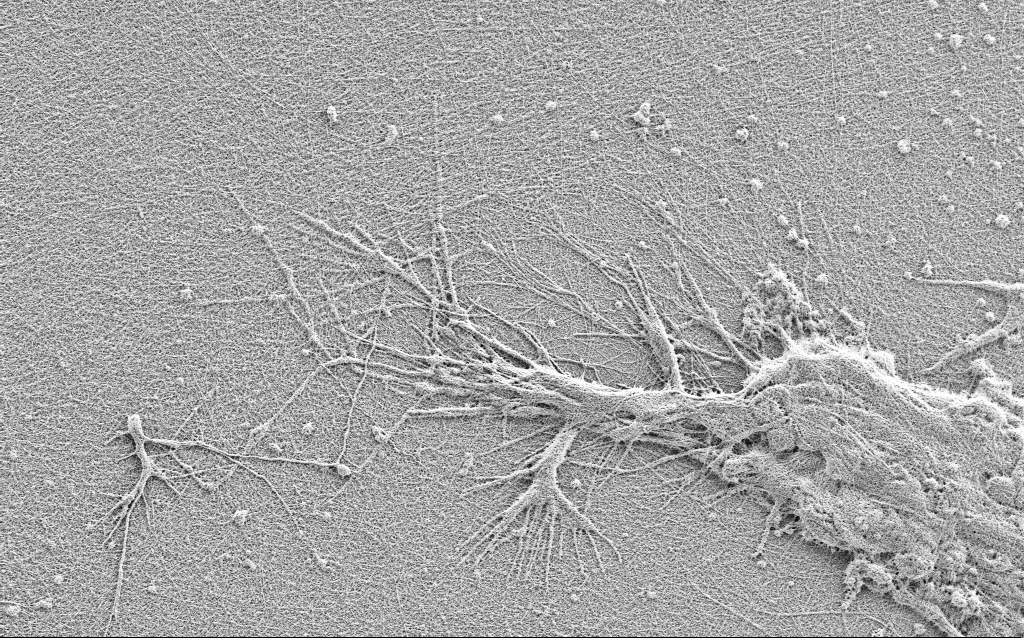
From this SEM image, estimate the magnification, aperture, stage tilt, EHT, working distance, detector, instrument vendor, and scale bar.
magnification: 7.5 K X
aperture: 30 µm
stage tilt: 0°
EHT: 0.9 kV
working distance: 3 mm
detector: SE2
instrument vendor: Zeiss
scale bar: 2000 nm